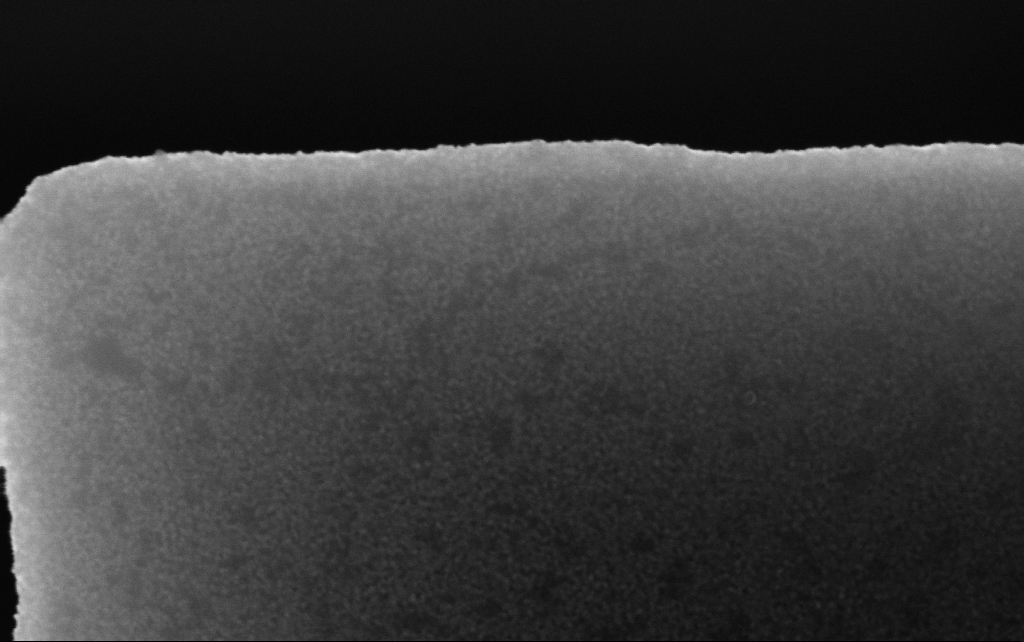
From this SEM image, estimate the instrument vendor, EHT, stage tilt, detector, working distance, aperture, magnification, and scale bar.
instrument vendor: Zeiss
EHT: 10 kV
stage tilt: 0°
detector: InLens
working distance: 2.5 mm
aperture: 30 µm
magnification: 200.15 K X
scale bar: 200 nm